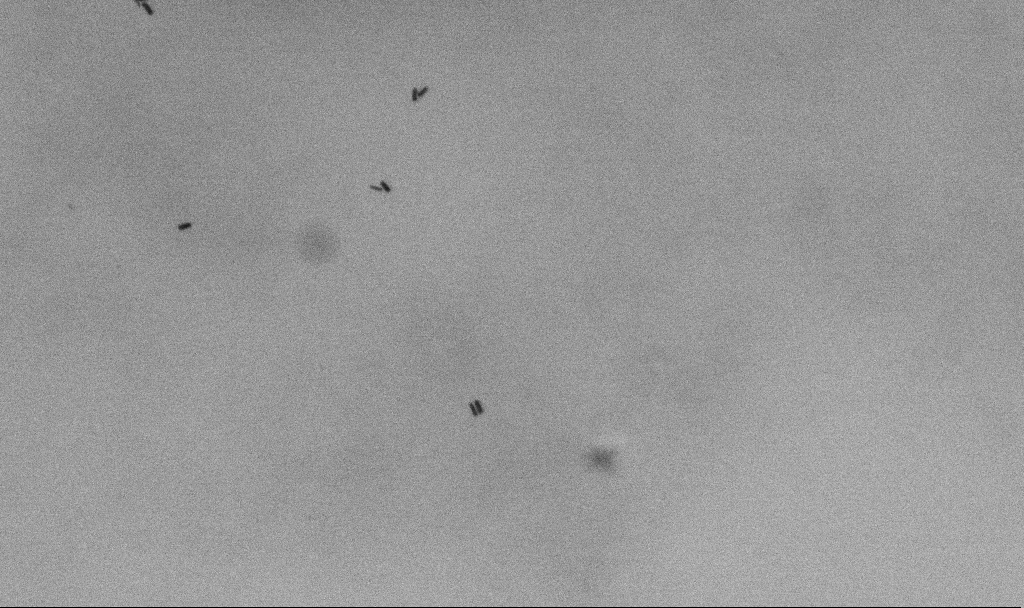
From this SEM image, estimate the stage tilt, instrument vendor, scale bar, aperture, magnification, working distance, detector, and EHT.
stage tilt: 0°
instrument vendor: Zeiss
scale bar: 1000 nm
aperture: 30 µm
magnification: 69.74 K X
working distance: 4.7 mm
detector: SE2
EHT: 5 kV